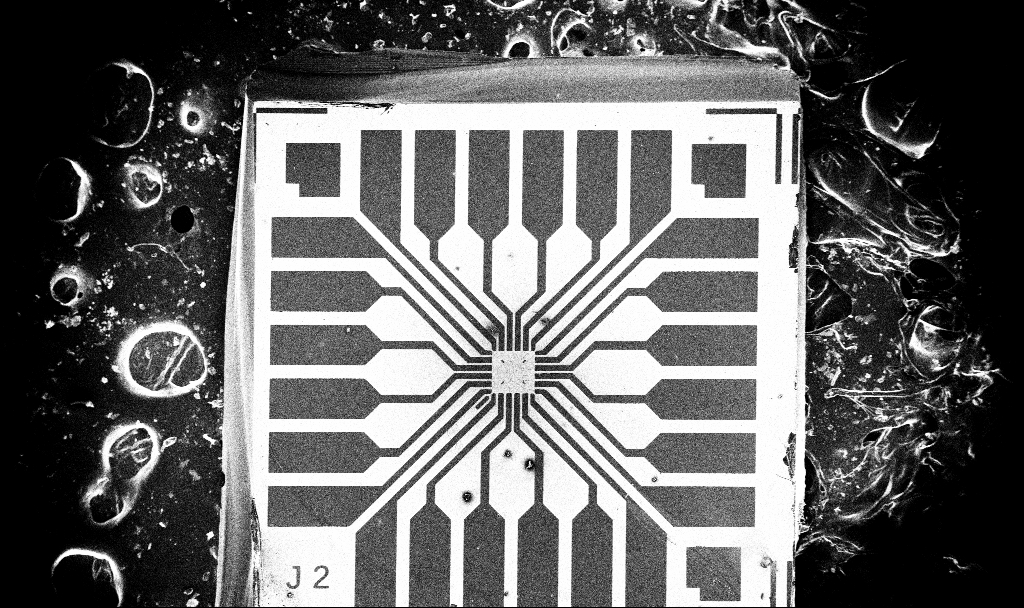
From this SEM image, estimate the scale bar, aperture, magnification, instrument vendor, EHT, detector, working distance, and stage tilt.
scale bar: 200000 nm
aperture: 30 µm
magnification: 0.1 K X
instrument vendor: Zeiss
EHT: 10 kV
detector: InLens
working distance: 6.7 mm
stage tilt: -0°